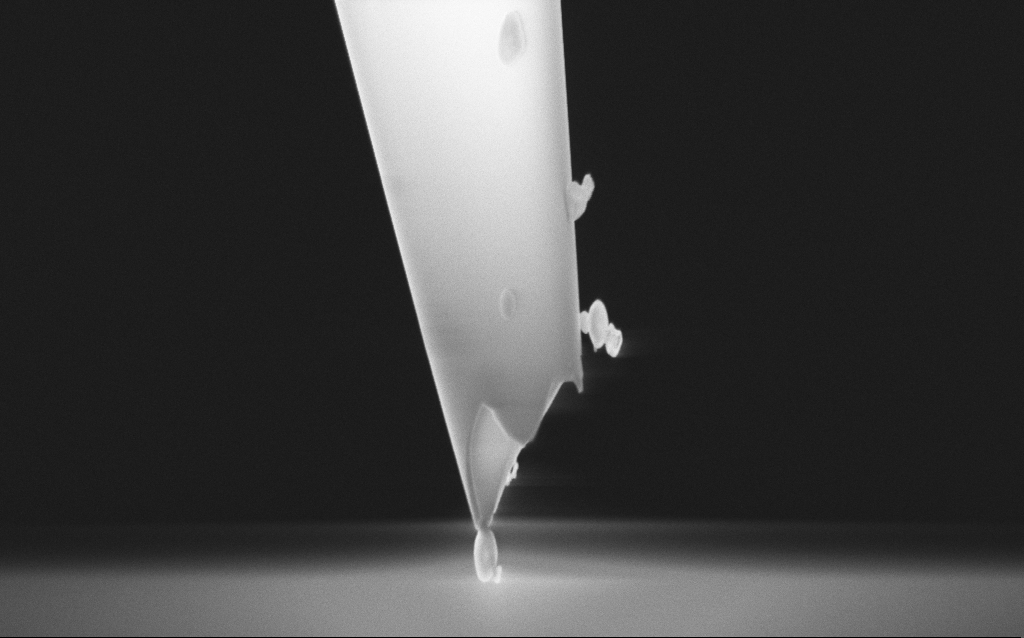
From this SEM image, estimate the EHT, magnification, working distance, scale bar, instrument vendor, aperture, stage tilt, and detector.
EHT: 5 kV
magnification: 47.87 K X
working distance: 3 mm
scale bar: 1000 nm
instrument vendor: Zeiss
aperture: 30 µm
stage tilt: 45°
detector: InLens